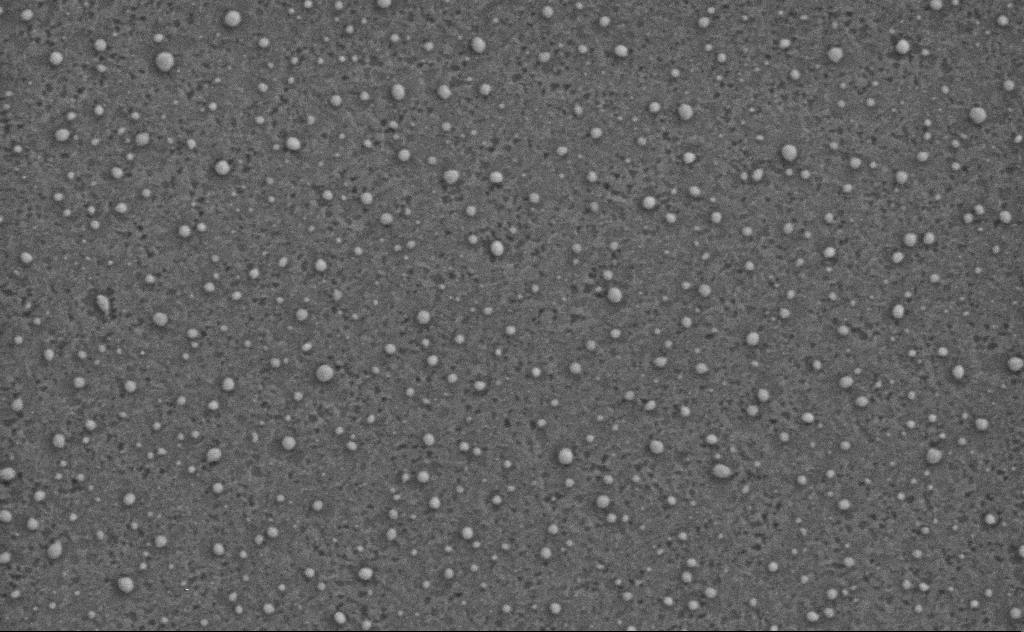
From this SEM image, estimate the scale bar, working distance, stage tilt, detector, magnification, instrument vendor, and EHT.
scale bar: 200 nm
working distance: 4 mm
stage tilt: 0°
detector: SE2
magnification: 80 K X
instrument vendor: Zeiss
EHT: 3 kV